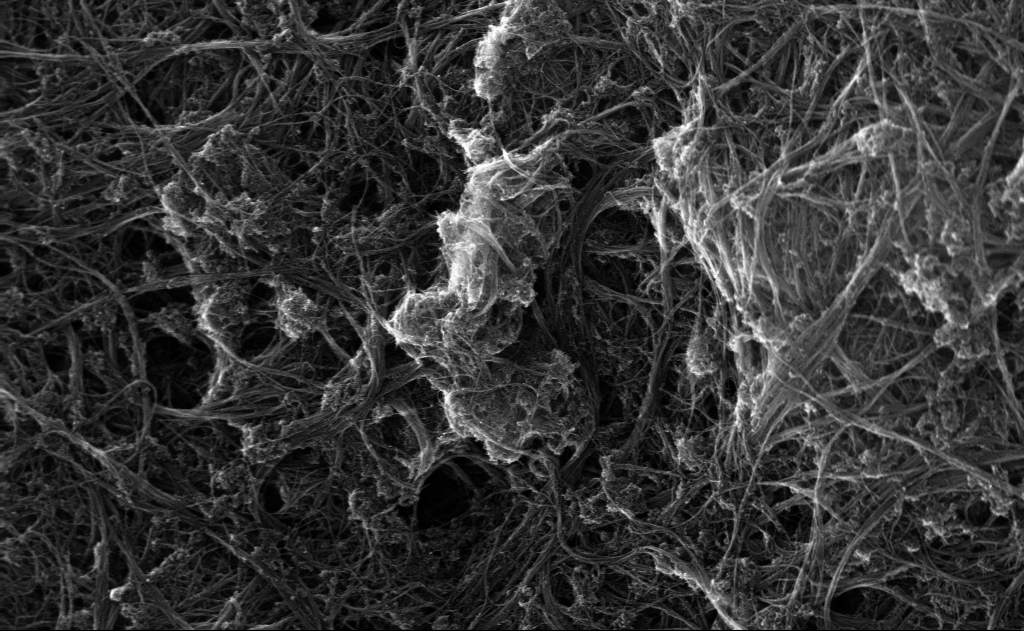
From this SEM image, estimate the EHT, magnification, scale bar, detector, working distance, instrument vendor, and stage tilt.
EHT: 10 kV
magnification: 68.23 K X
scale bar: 1000 nm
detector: InLens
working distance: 3 mm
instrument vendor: Zeiss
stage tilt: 0°